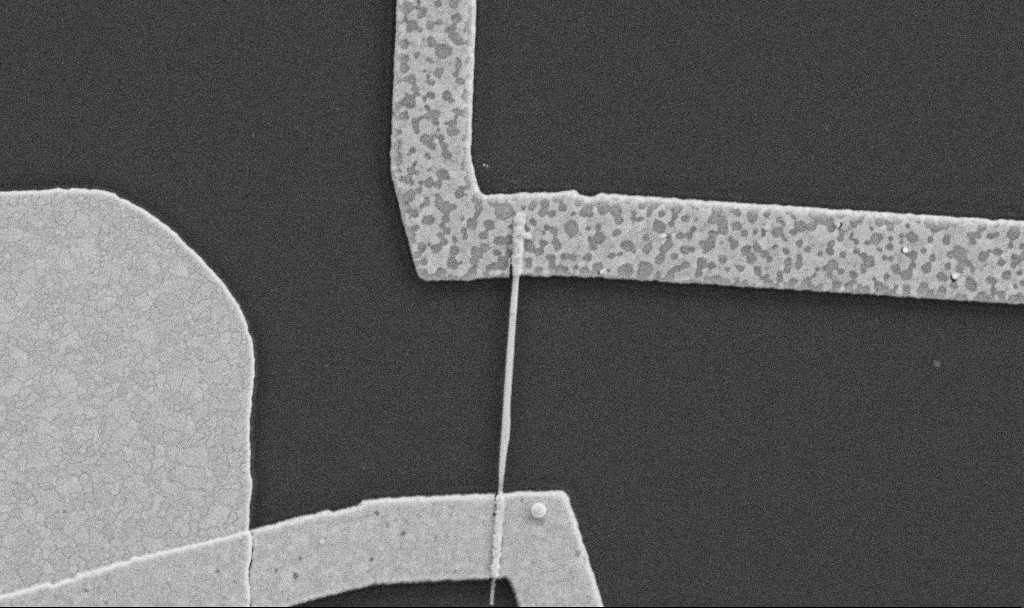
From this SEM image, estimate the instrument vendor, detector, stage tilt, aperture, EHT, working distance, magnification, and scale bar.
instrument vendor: Zeiss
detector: SE2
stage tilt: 0°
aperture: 30 µm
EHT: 5 kV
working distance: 8.7 mm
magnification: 30 K X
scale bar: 1000 nm